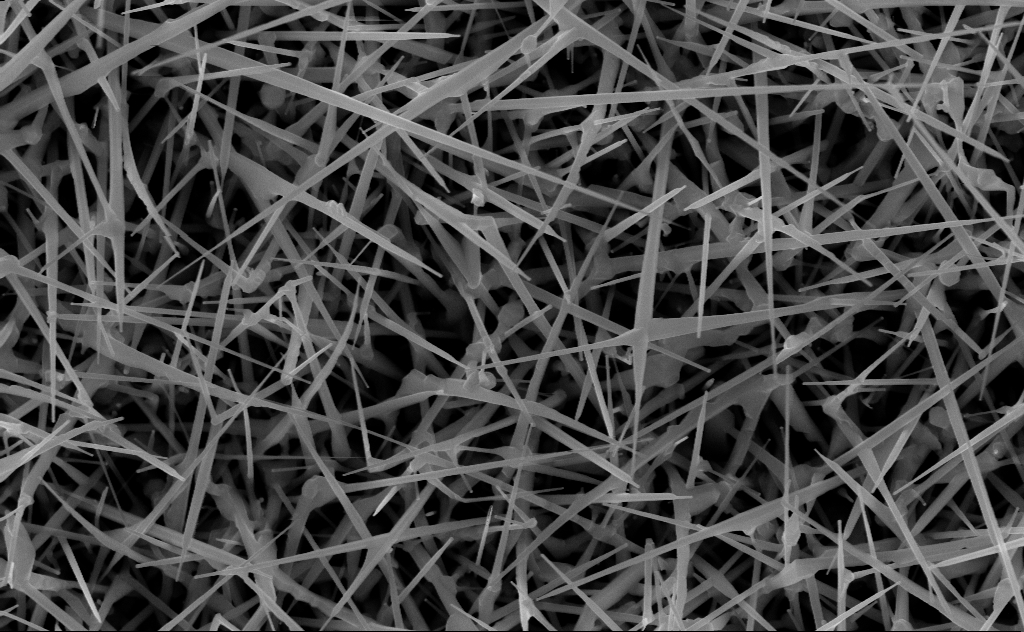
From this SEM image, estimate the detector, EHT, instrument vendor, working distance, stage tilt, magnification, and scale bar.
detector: InLens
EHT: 10 kV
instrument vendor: Zeiss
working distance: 10 mm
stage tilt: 0°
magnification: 40 K X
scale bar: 1000 nm